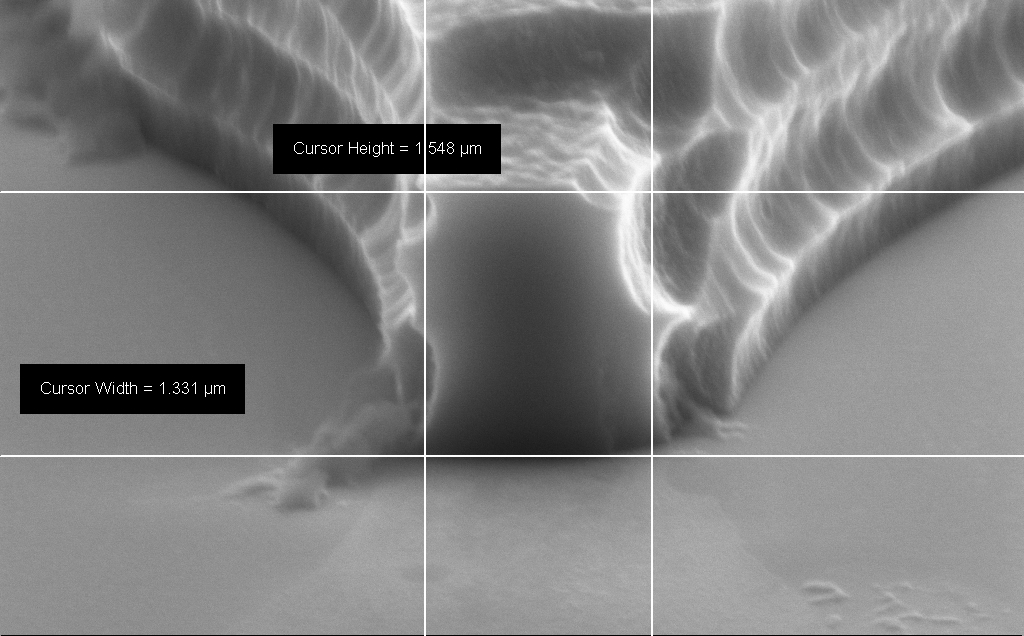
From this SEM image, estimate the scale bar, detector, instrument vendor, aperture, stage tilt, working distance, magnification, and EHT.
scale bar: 1000 nm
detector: SE2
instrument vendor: Zeiss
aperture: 30 µm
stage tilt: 70°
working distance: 12 mm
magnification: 62.61 K X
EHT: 8 kV